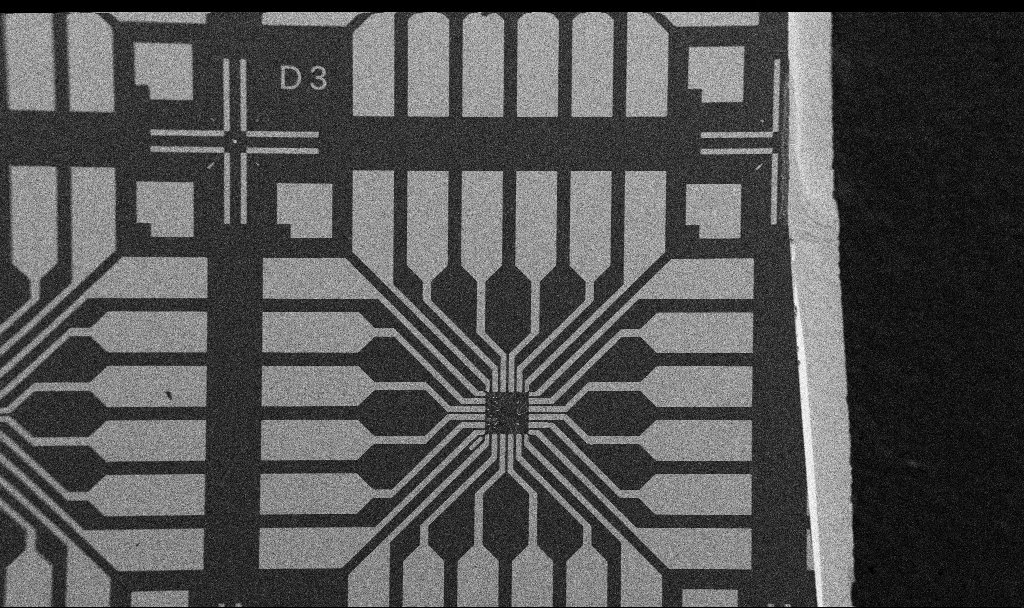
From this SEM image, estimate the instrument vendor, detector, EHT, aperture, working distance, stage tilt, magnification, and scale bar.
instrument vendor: Zeiss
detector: SE2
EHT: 5 kV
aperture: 30 µm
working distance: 10.7 mm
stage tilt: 0°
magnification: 0.1 K X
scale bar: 200000 nm